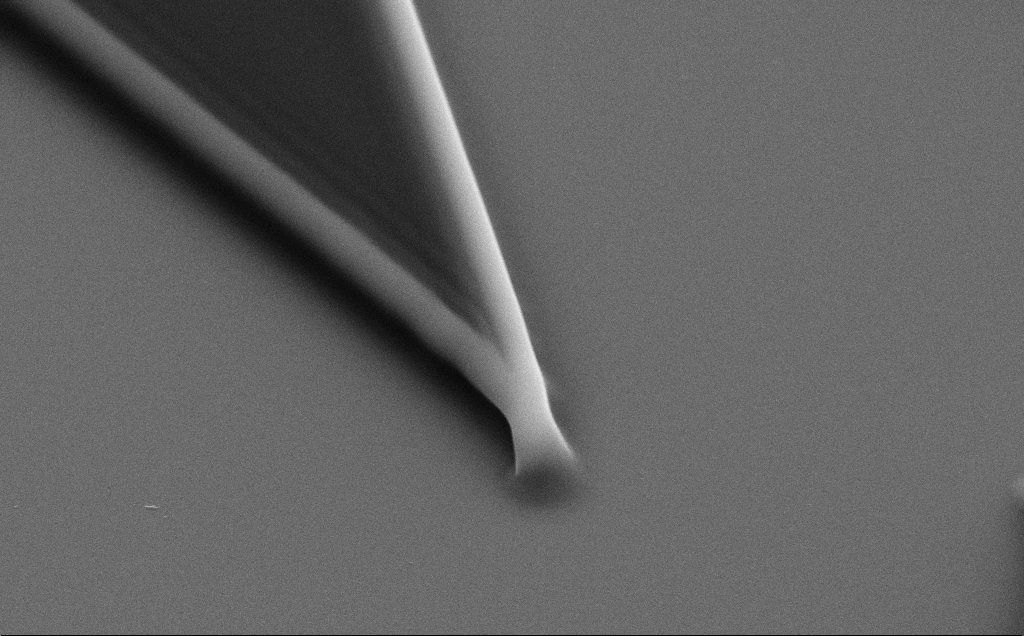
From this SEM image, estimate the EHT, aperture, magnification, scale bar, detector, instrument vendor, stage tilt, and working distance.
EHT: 10 kV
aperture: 30 µm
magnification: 14.74 K X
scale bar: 2000 nm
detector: SE2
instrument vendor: Zeiss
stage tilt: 35°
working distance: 8 mm